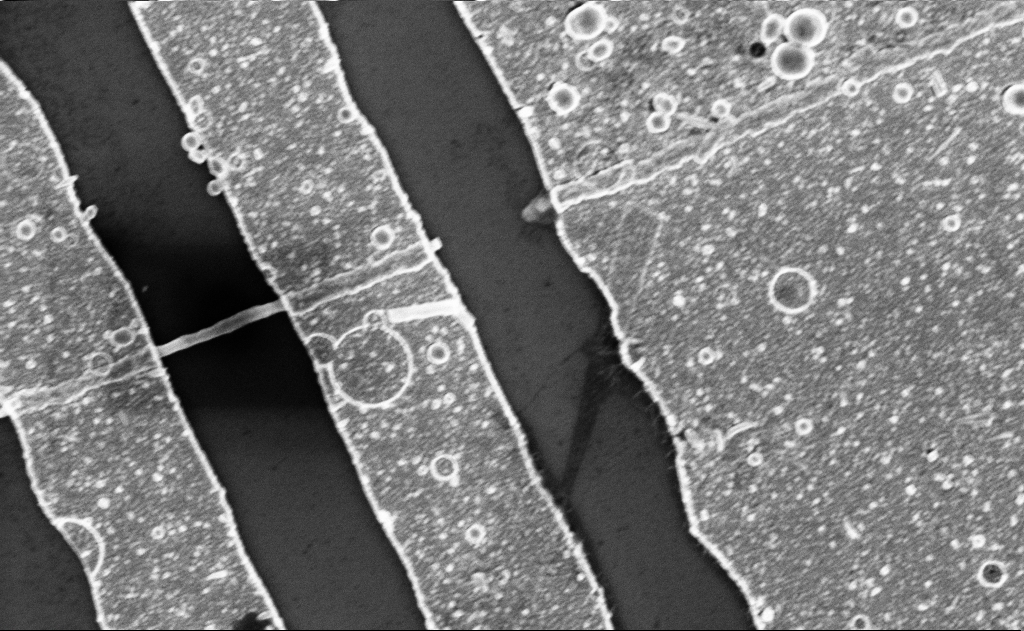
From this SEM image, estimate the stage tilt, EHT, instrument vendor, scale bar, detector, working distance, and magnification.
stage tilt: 0°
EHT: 5 kV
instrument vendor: Zeiss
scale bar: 1000 nm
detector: InLens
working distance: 7 mm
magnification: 26.93 K X